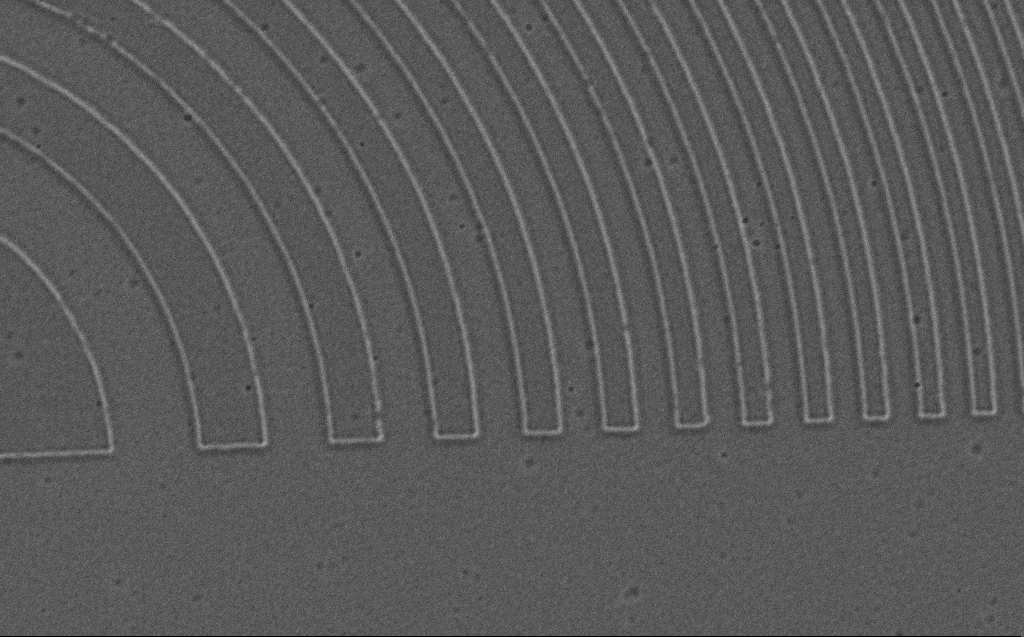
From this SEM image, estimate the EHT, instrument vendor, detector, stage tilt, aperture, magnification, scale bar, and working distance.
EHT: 3 kV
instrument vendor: Zeiss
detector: SE2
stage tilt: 0°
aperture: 30 µm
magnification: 23.46 K X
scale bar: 1000 nm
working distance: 4 mm